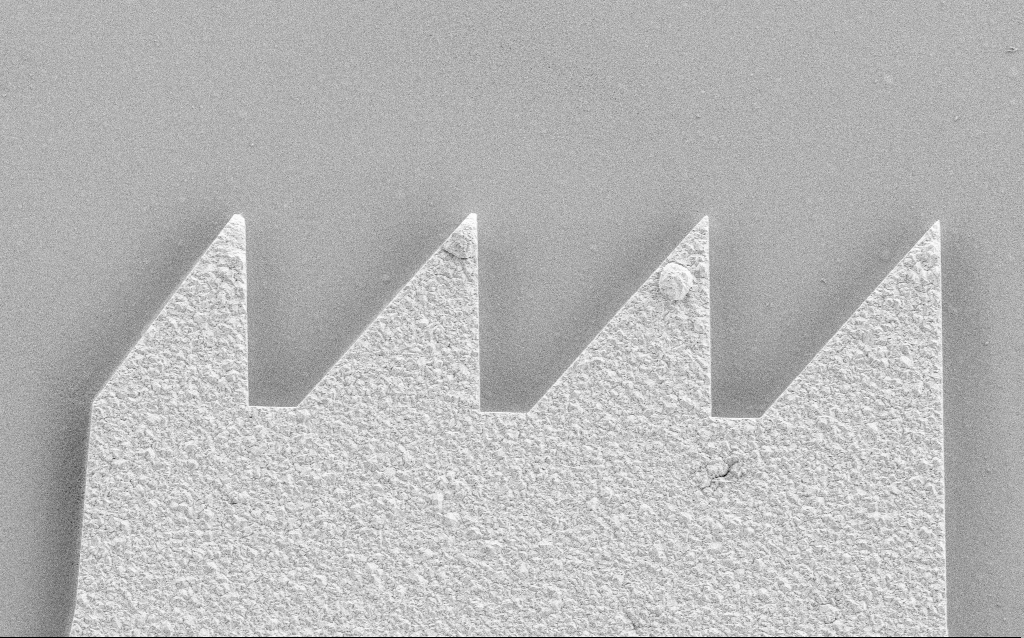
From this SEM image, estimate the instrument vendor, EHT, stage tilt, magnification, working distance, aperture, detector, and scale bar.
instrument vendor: Zeiss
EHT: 3 kV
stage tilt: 0°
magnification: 7.52 K X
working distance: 4.5 mm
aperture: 30 µm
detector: SE2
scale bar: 2000 nm